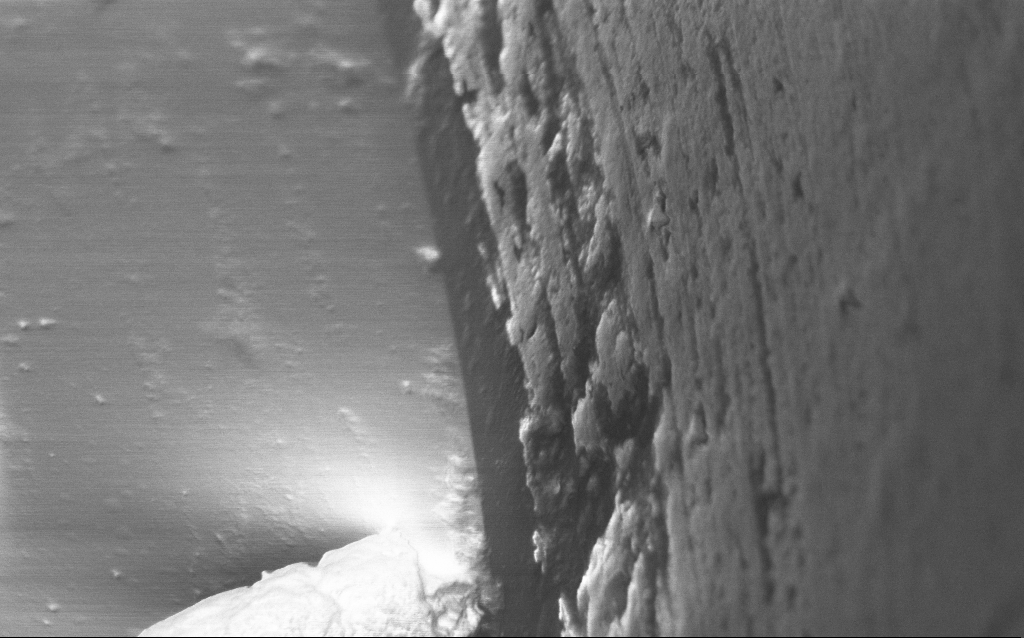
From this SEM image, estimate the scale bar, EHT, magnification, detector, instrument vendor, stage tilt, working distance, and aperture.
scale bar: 1000 nm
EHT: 1 kV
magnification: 25 K X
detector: InLens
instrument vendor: Zeiss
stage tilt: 45°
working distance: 5 mm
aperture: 30 µm